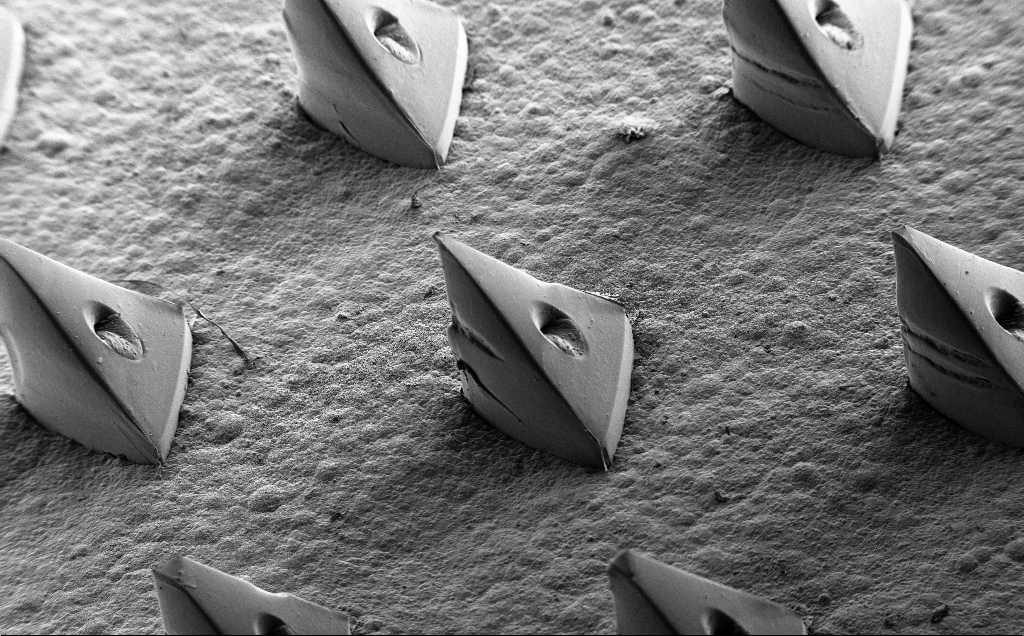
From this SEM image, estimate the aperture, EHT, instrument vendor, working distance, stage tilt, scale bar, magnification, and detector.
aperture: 30 µm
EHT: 5 kV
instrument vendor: Zeiss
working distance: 12 mm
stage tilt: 35°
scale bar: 200000 nm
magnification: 0.11 K X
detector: SE2